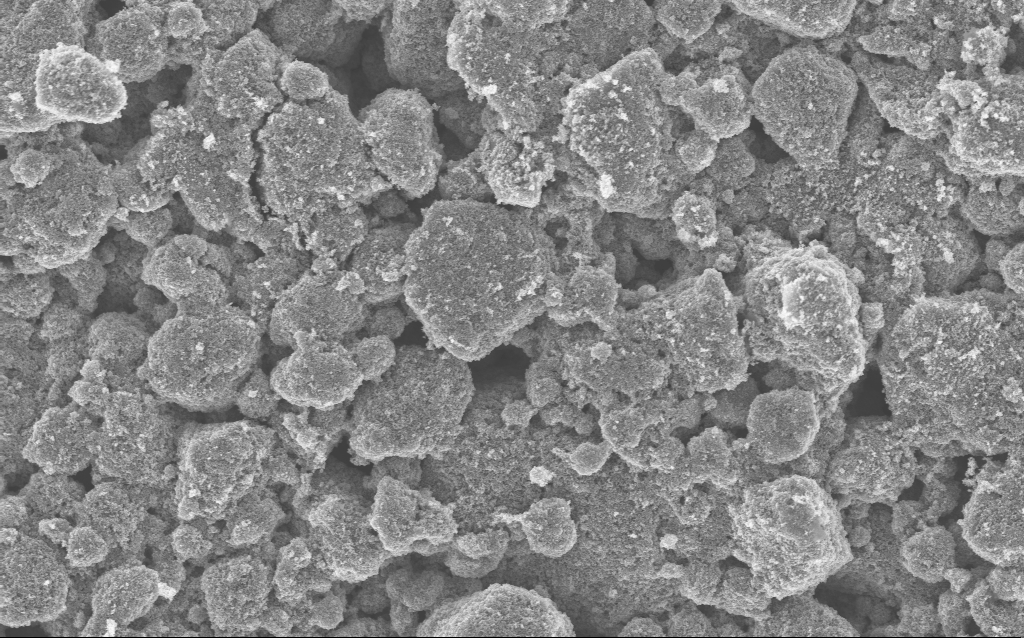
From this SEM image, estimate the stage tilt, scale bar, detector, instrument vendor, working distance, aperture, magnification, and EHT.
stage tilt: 0°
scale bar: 1000 nm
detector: InLens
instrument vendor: Zeiss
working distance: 4.7 mm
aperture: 30 µm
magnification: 12.08 K X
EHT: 5 kV